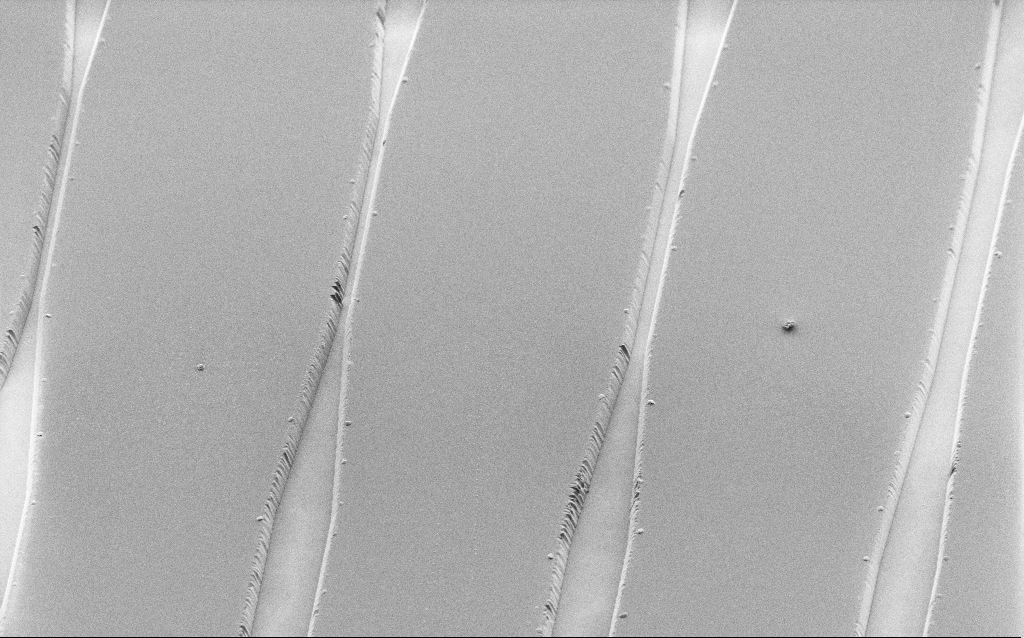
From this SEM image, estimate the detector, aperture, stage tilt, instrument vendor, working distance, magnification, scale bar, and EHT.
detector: SE2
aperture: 30 µm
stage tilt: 45°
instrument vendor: Zeiss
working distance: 8 mm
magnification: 1.06 K X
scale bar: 20000 nm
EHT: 1.2 kV